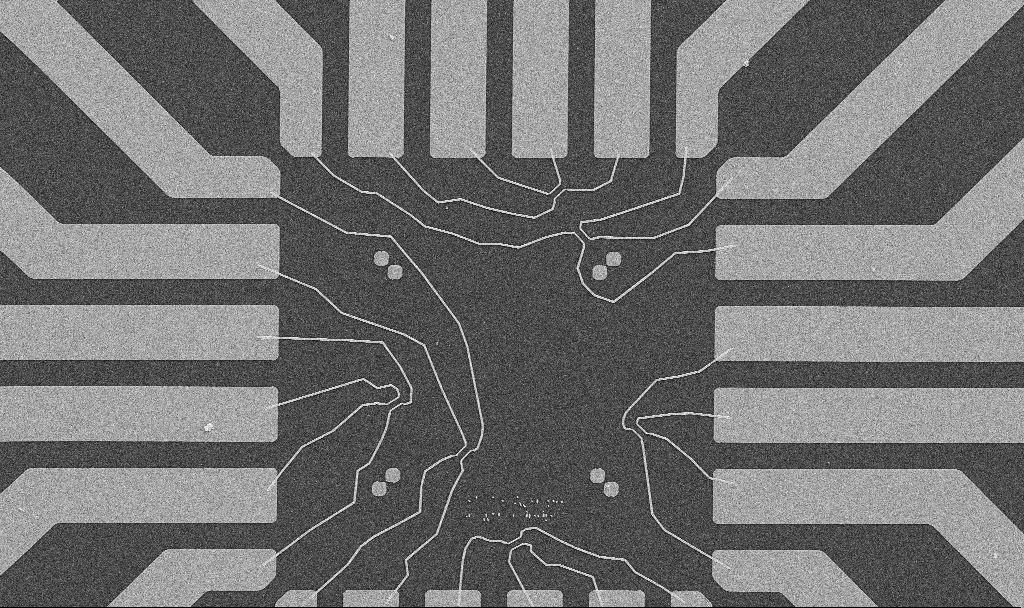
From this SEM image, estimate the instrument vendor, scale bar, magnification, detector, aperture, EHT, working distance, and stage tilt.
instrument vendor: Zeiss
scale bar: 20000 nm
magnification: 1 K X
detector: SE2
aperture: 30 µm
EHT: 10 kV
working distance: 10.7 mm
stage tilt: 0°